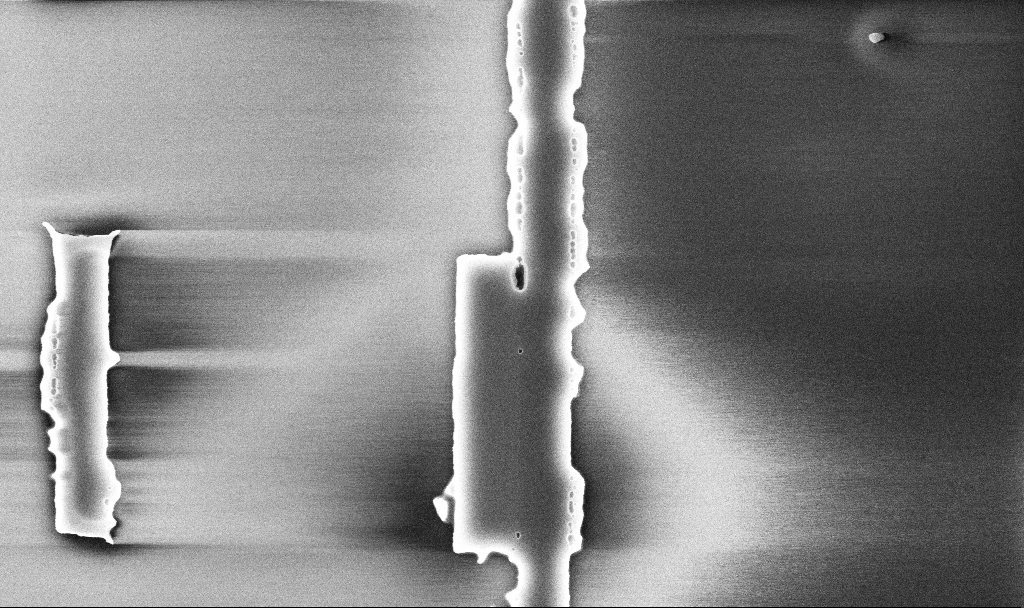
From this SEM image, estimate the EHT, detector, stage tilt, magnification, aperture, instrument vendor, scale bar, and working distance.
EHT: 5 kV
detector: InLens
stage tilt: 0°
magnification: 36.61 K X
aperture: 30 µm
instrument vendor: Zeiss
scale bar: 1000 nm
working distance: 10.1 mm